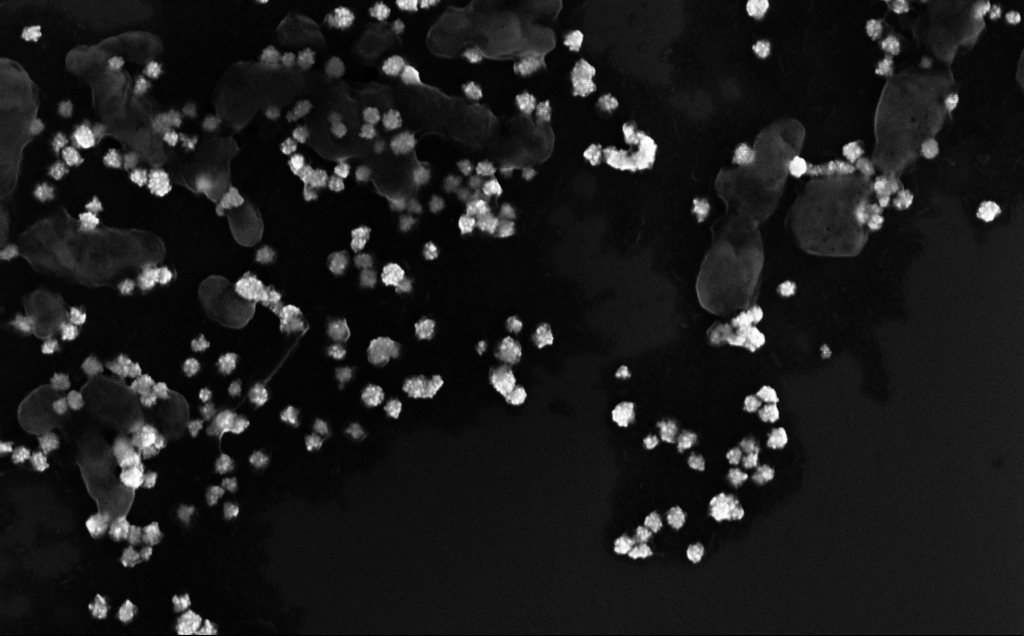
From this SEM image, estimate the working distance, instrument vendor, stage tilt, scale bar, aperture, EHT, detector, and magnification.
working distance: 4 mm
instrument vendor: Zeiss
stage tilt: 0°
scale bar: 200 nm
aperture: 30 µm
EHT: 10 kV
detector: InLens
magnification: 107.01 K X